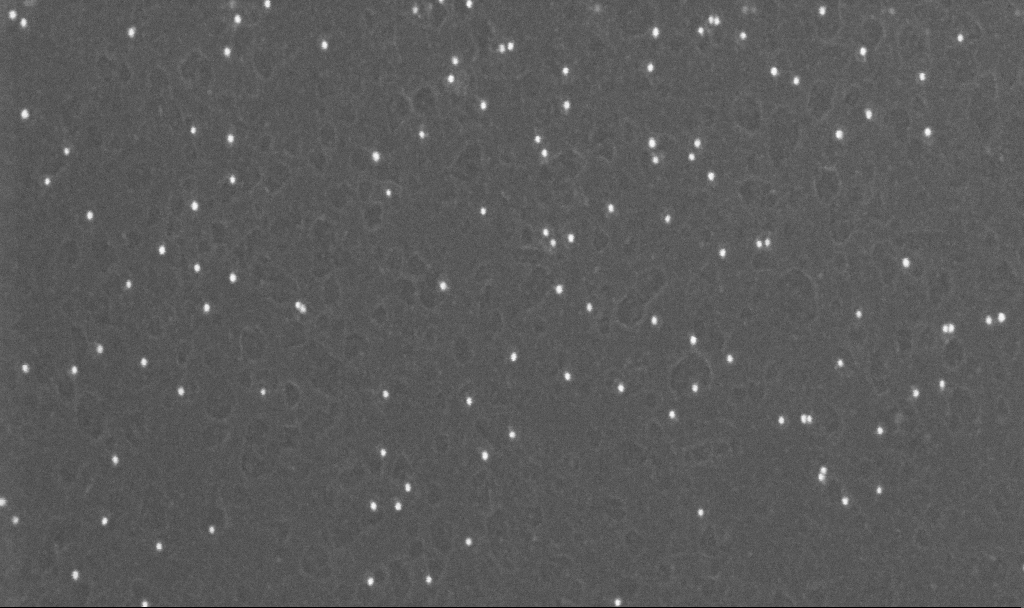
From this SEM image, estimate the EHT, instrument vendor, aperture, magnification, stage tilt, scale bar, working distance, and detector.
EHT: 10 kV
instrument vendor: Zeiss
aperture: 30 µm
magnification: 200 K X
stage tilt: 0°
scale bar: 100 nm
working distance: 3.1 mm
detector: InLens